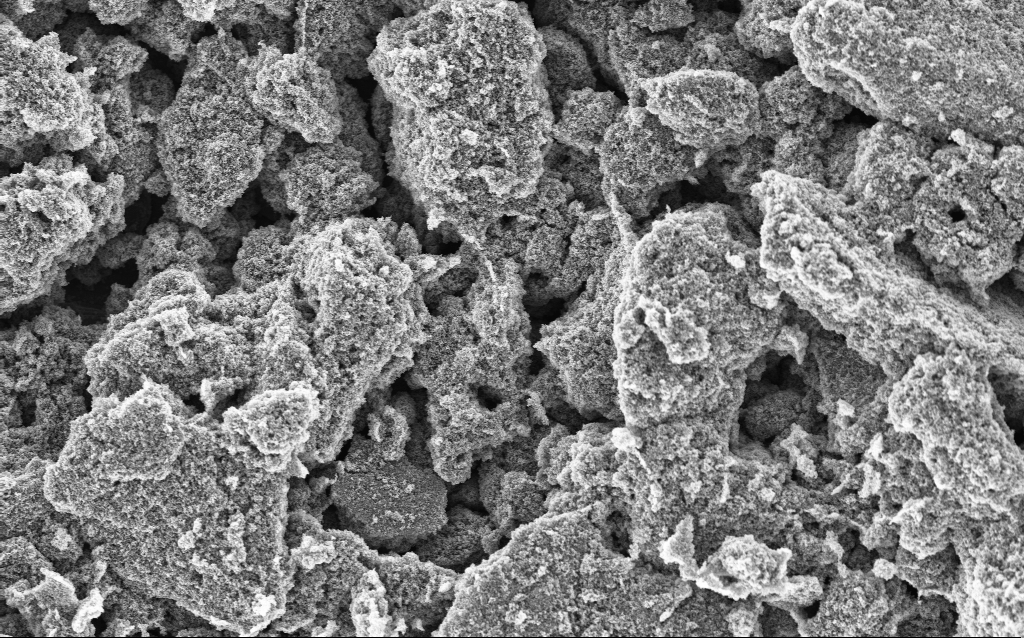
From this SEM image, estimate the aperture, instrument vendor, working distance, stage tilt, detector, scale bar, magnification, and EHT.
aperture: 30 µm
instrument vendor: Zeiss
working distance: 4.4 mm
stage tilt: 0°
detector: InLens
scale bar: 10000 nm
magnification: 6.42 K X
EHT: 5 kV